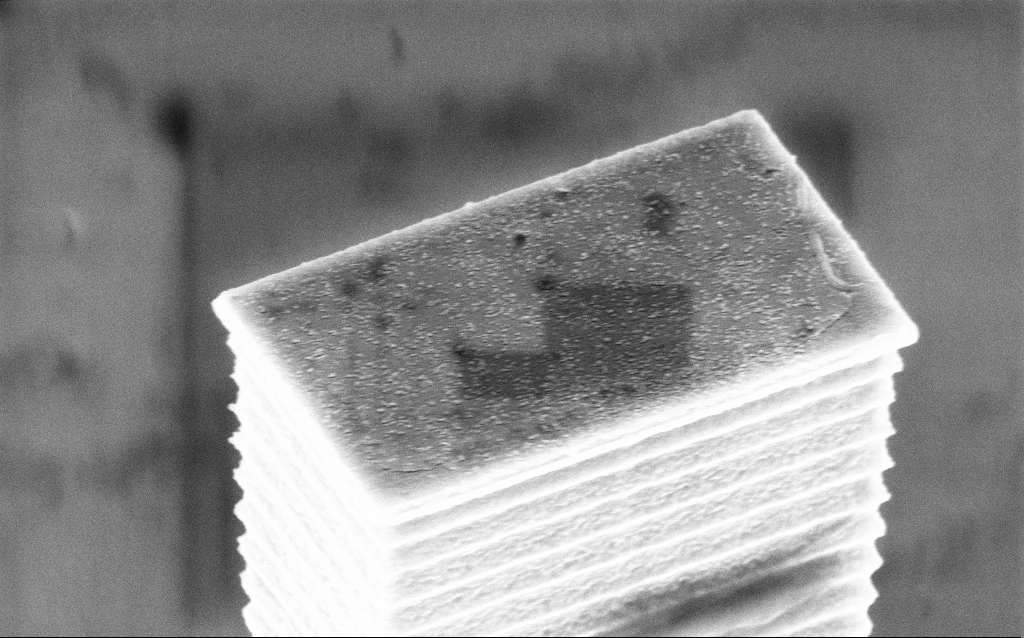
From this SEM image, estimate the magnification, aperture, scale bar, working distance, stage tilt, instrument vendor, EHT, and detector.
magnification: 46.08 K X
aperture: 30 µm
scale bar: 1000 nm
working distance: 4 mm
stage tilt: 45°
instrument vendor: Zeiss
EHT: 5 kV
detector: InLens